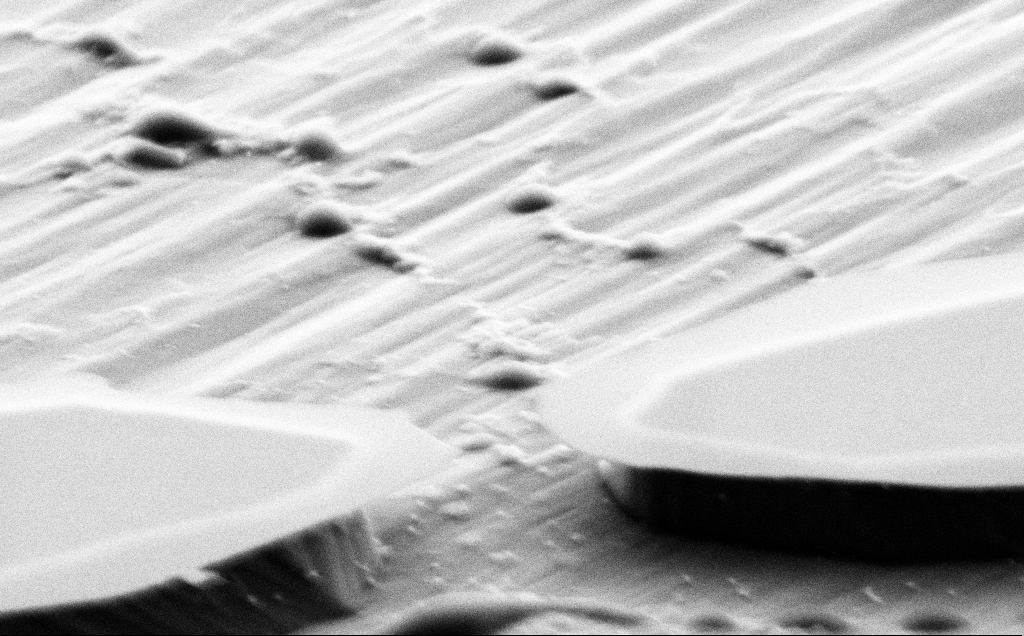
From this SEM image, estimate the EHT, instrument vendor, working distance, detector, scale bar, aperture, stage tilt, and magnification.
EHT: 5 kV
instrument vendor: Zeiss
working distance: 7 mm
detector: SE2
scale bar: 2000 nm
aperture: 30 µm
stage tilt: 70°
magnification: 21.19 K X